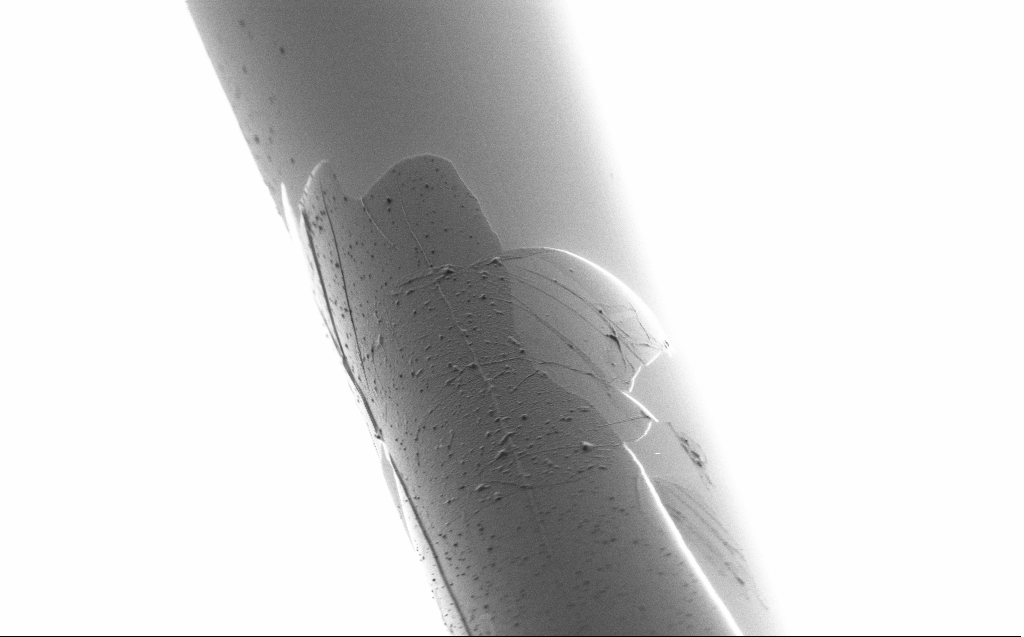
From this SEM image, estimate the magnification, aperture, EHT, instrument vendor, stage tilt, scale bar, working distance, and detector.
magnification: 5 K X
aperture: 30 µm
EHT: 1 kV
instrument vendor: Zeiss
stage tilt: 45°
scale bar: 10000 nm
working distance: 4 mm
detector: SE2